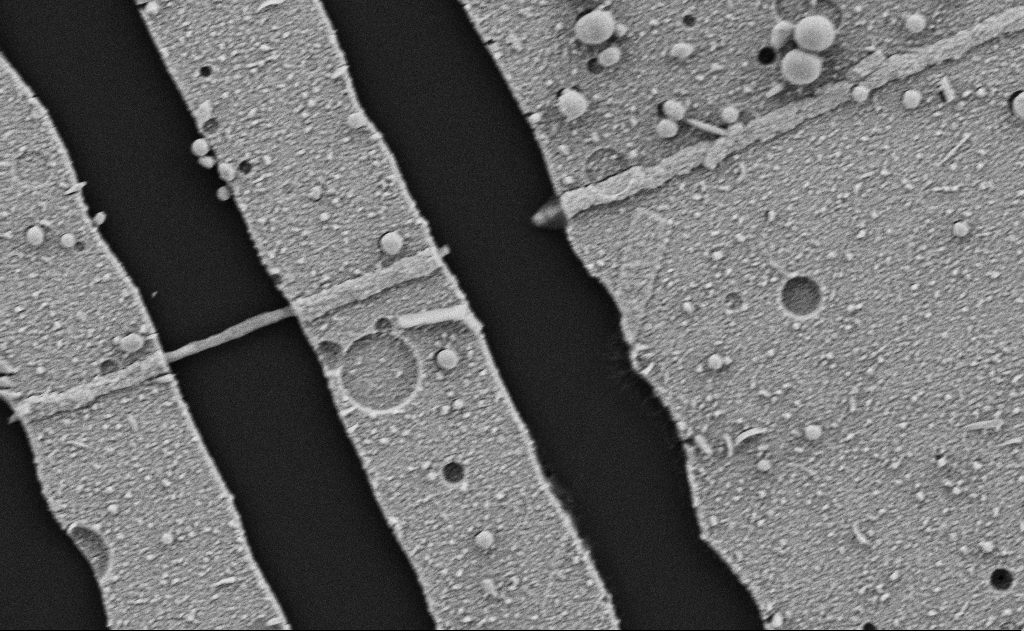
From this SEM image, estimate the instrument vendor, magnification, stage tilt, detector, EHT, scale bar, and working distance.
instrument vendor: Zeiss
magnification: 26.93 K X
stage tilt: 0°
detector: SE2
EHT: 5 kV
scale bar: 1000 nm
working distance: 7 mm